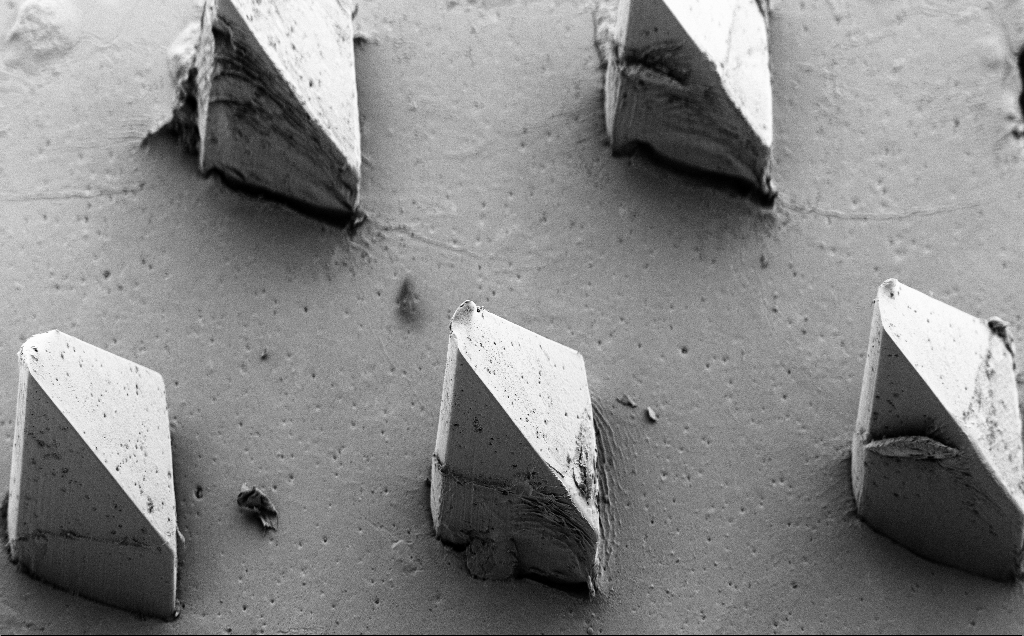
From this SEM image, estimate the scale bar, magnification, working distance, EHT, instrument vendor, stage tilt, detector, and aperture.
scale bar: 100000 nm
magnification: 0.168 K X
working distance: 8 mm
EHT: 5 kV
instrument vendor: Zeiss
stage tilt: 40°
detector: SE2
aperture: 30 µm